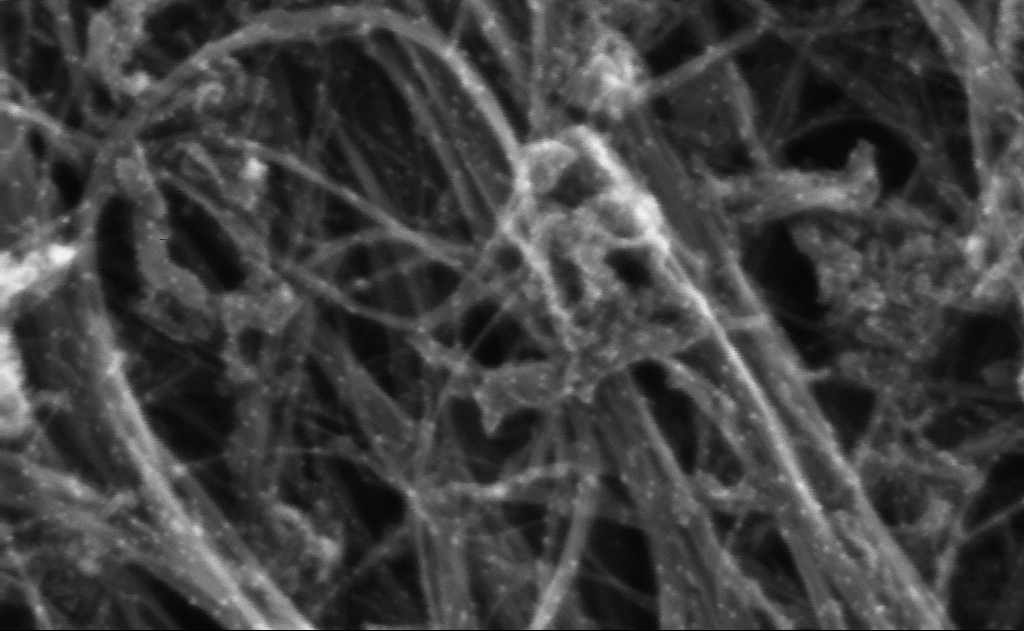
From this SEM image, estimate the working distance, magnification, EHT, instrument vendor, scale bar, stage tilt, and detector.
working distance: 3 mm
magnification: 731.48 K X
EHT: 10 kV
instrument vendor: Zeiss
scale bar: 20 nm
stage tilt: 0°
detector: InLens